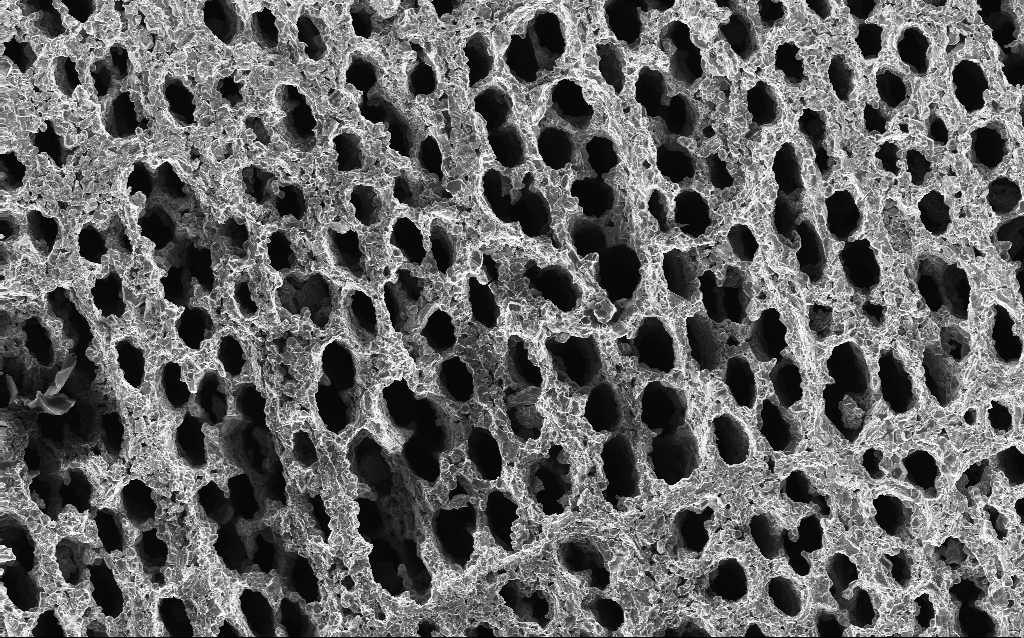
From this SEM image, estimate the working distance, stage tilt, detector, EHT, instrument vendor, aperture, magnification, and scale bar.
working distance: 3 mm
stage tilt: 0°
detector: InLens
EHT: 10 kV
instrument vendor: Zeiss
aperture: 30 µm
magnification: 0.5 K X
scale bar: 100000 nm